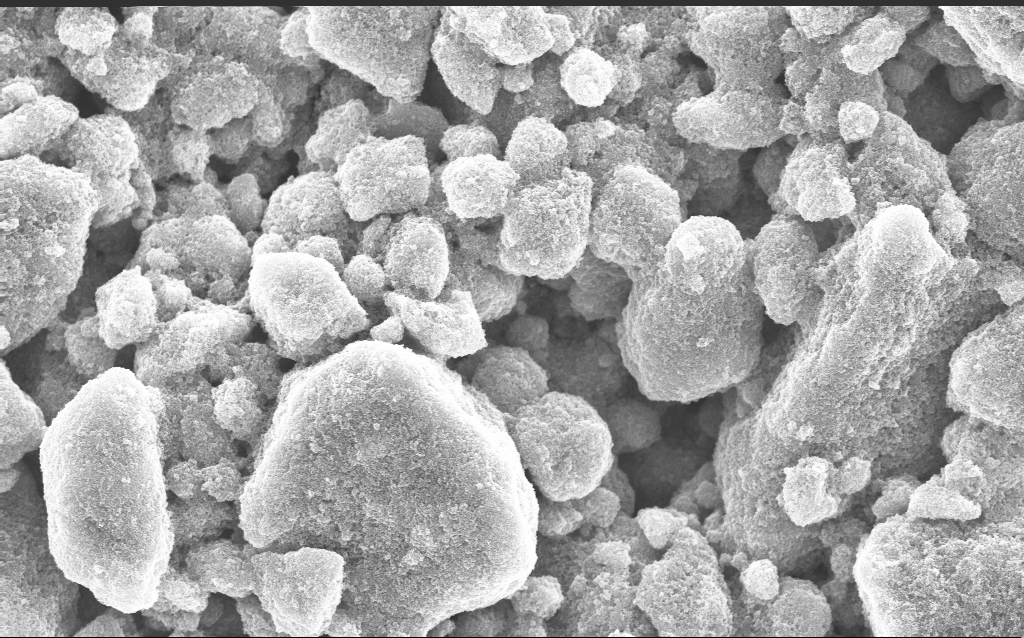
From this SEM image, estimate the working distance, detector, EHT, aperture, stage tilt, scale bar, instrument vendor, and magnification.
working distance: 3.6 mm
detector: InLens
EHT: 10 kV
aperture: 30 µm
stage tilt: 0°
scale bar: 2000 nm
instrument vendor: Zeiss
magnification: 14.54 K X